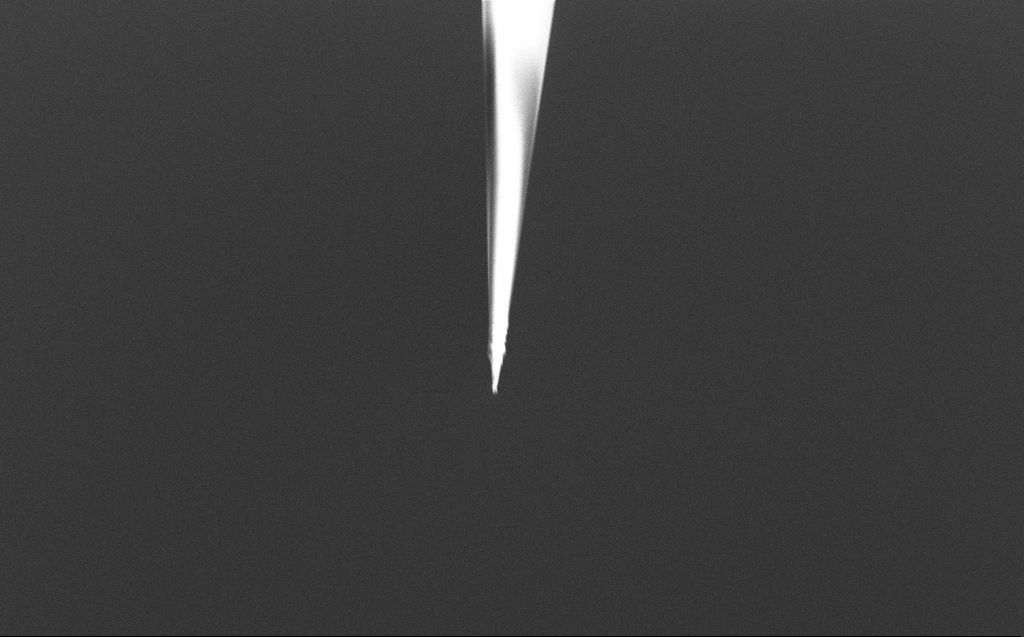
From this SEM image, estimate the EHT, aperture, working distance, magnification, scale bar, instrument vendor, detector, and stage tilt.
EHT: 5 kV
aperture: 20 µm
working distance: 5 mm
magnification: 3.33 K X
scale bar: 20000 nm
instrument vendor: Zeiss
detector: InLens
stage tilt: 45°